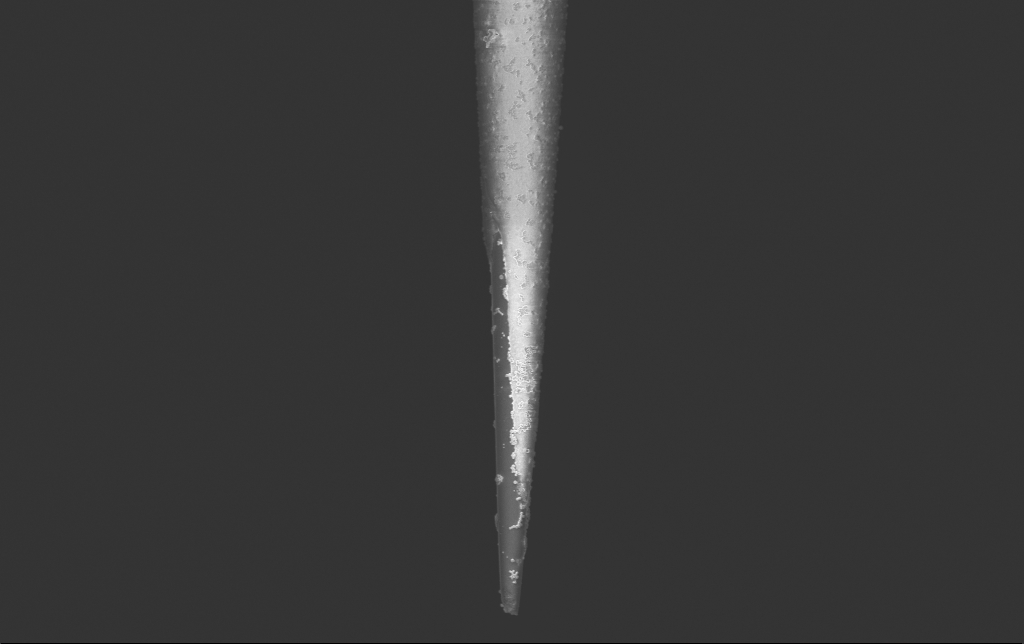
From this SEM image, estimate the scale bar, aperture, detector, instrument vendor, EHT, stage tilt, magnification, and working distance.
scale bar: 2000 nm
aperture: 30 µm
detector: InLens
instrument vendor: Zeiss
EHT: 2 kV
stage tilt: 0°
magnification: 10 K X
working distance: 5.9 mm